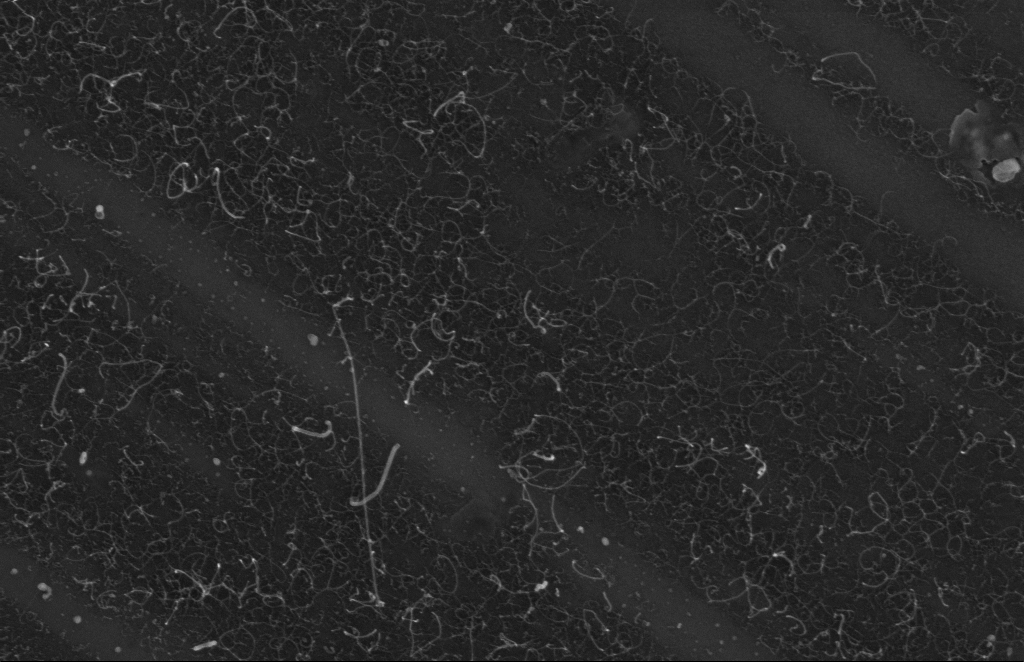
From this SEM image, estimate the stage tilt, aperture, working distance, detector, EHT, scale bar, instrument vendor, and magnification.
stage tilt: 0°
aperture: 20 µm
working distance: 5 mm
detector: InLens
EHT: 5 kV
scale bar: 200 nm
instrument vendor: Zeiss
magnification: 77.13 K X